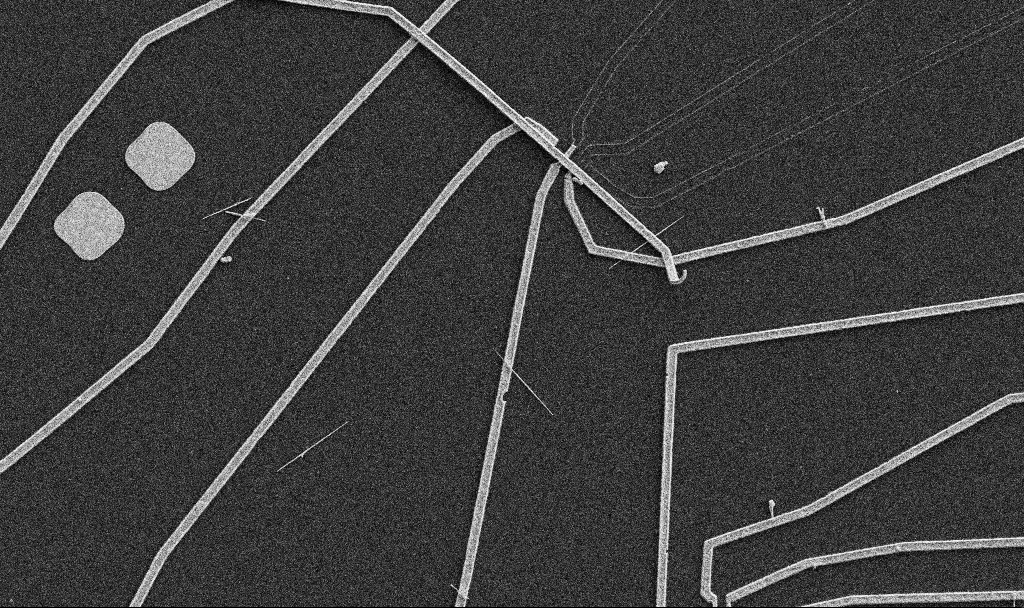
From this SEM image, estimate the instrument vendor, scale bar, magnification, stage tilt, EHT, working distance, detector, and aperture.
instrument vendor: Zeiss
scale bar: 10000 nm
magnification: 5 K X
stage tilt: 0°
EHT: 5 kV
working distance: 10.7 mm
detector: SE2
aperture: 30 µm